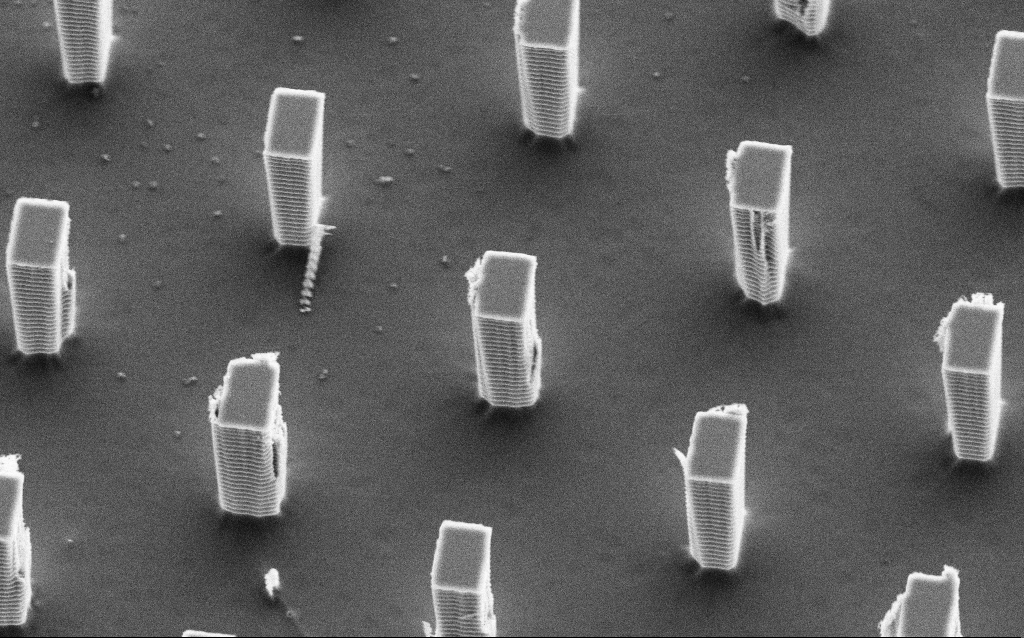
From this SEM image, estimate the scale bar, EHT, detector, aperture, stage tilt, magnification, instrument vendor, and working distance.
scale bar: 2000 nm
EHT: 5 kV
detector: SE2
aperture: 30 µm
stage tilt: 28.6°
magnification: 9.64 K X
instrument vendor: Zeiss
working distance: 11.6 mm